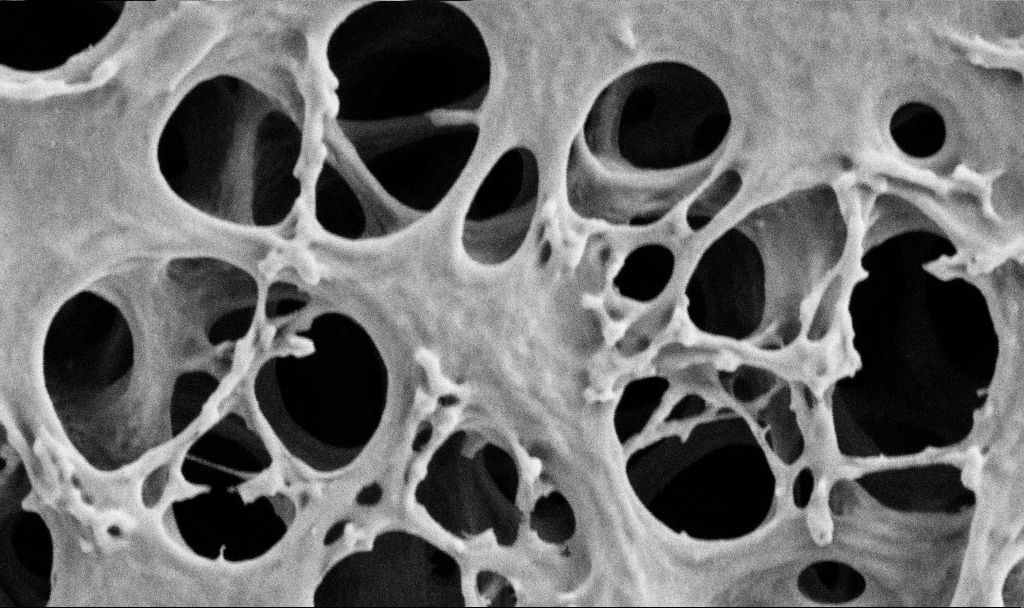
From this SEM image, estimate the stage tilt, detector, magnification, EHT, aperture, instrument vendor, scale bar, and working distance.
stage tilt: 0°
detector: SE2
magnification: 50 K X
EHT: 2 kV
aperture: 30 µm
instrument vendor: Zeiss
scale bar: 1000 nm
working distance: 3.5 mm